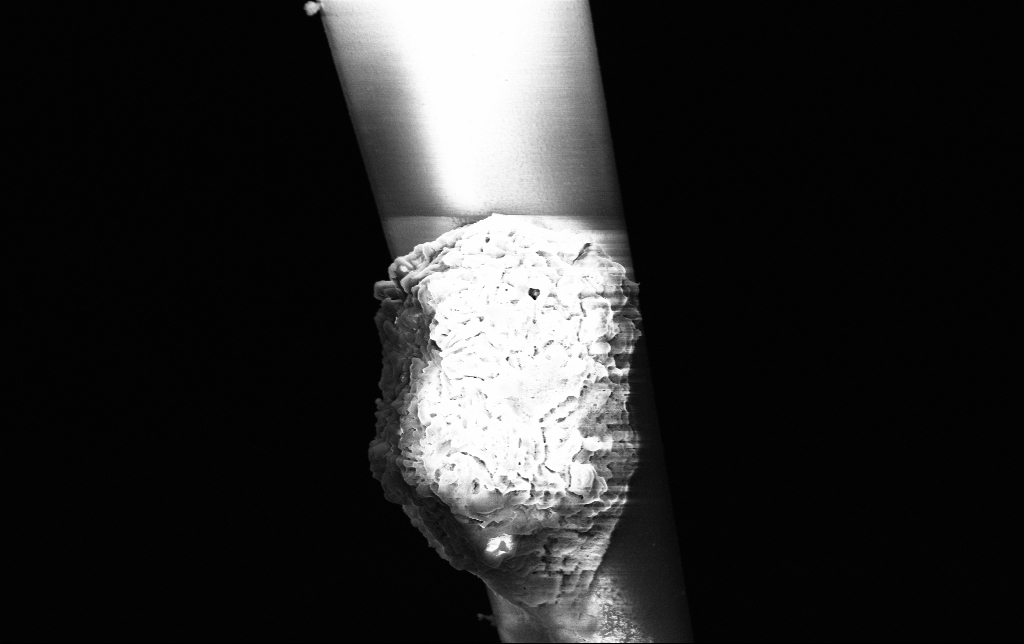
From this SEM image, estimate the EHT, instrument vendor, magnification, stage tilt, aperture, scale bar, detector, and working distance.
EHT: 3 kV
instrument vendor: Zeiss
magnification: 15 K X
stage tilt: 0°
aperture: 30 µm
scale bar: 2000 nm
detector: InLens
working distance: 6.5 mm